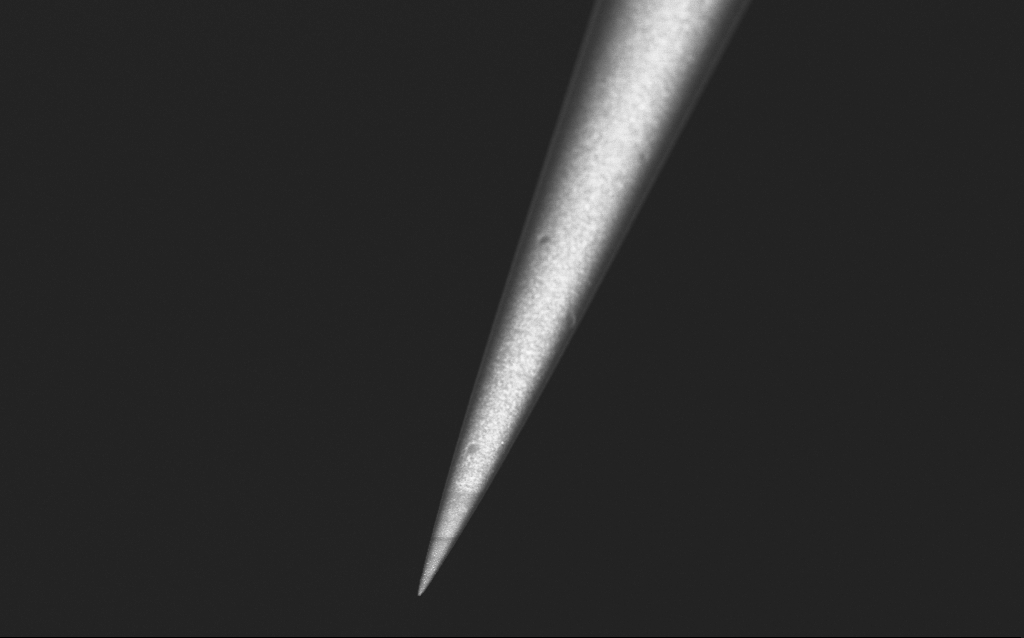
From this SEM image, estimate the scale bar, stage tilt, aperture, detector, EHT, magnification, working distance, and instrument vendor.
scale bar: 2000 nm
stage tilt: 45°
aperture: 30 µm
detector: InLens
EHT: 2.5 kV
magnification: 10 K X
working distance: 5 mm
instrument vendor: Zeiss